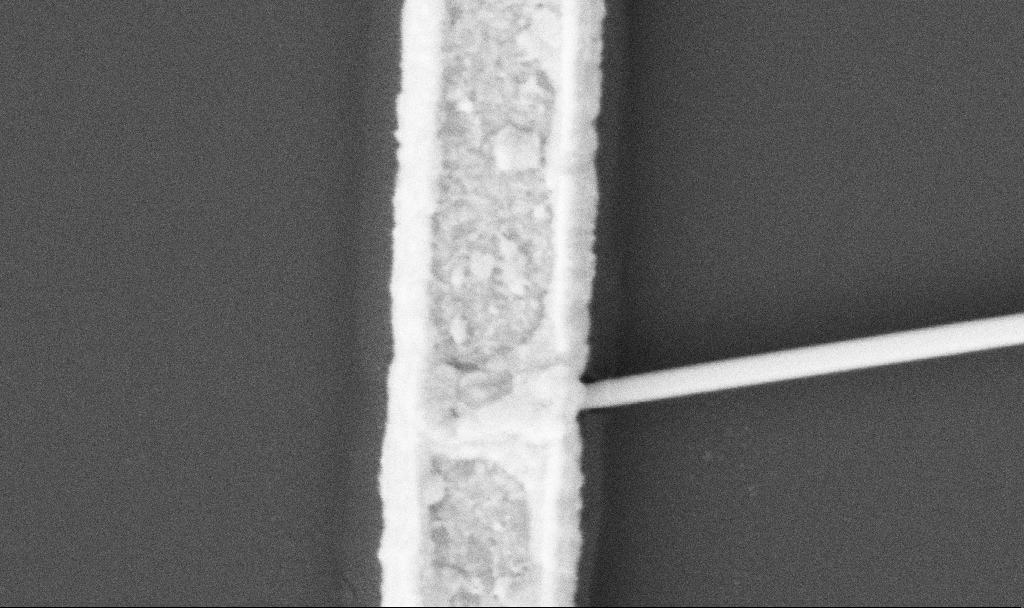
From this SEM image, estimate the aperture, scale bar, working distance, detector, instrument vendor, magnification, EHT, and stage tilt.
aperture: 30 µm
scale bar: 200 nm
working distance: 10.7 mm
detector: SE2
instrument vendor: Zeiss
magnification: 100 K X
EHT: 5 kV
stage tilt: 0°